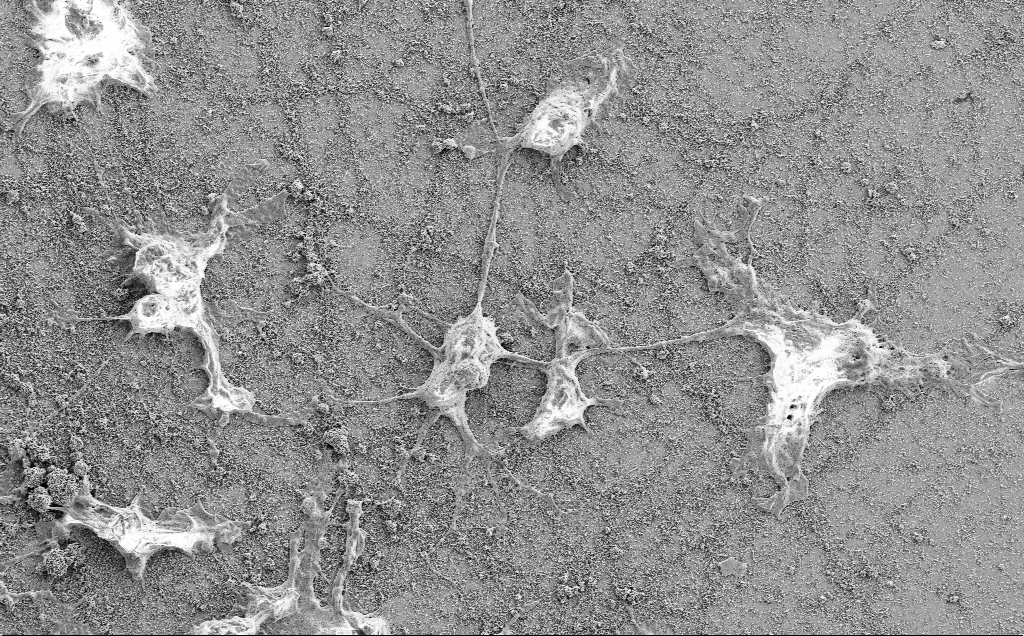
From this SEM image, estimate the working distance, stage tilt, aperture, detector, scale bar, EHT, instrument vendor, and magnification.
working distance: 7.1 mm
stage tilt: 0°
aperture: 30 µm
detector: SE2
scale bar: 10000 nm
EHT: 2 kV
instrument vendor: Zeiss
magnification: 2.5 K X